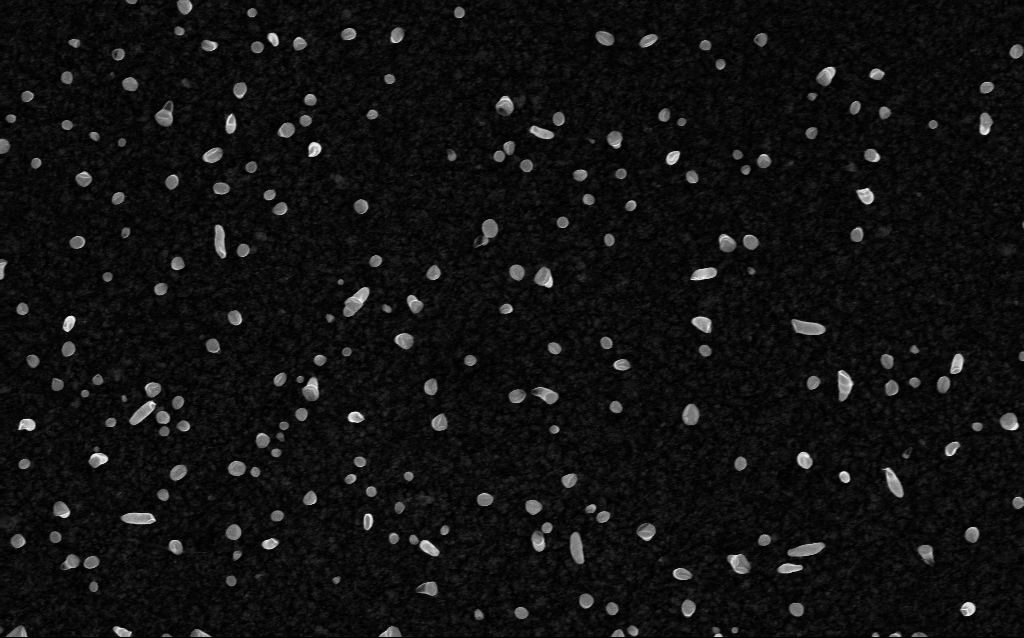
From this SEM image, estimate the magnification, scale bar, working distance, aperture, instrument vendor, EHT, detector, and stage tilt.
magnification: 50 K X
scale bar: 1000 nm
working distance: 2 mm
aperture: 30 µm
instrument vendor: Zeiss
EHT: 5 kV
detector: InLens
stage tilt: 0°